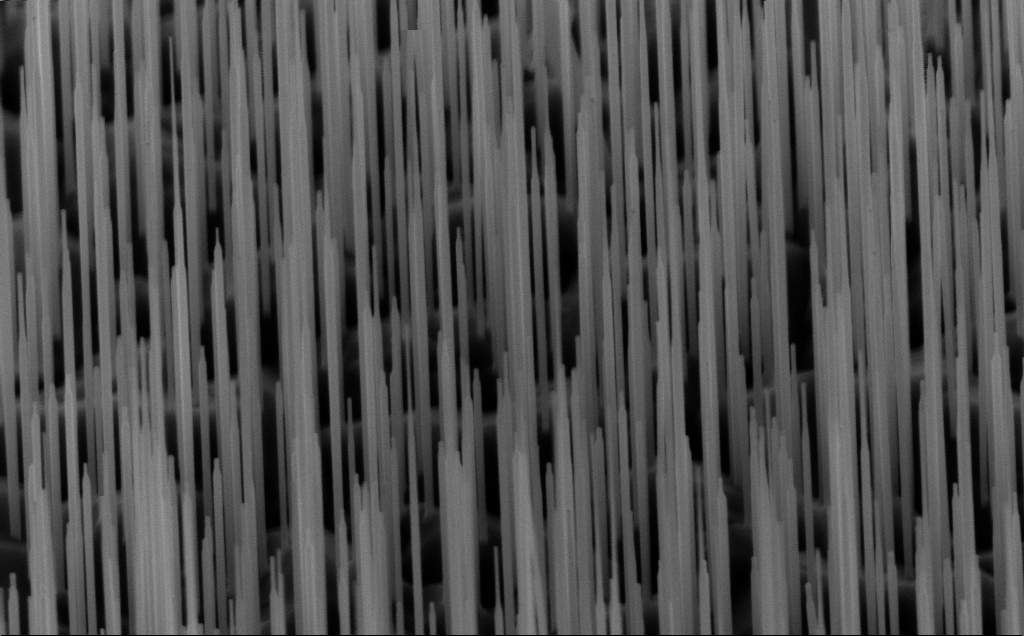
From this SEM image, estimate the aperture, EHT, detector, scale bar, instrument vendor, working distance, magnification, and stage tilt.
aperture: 30 µm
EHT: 10 kV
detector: InLens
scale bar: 1000 nm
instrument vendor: Zeiss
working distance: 6 mm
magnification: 40 K X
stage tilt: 45°